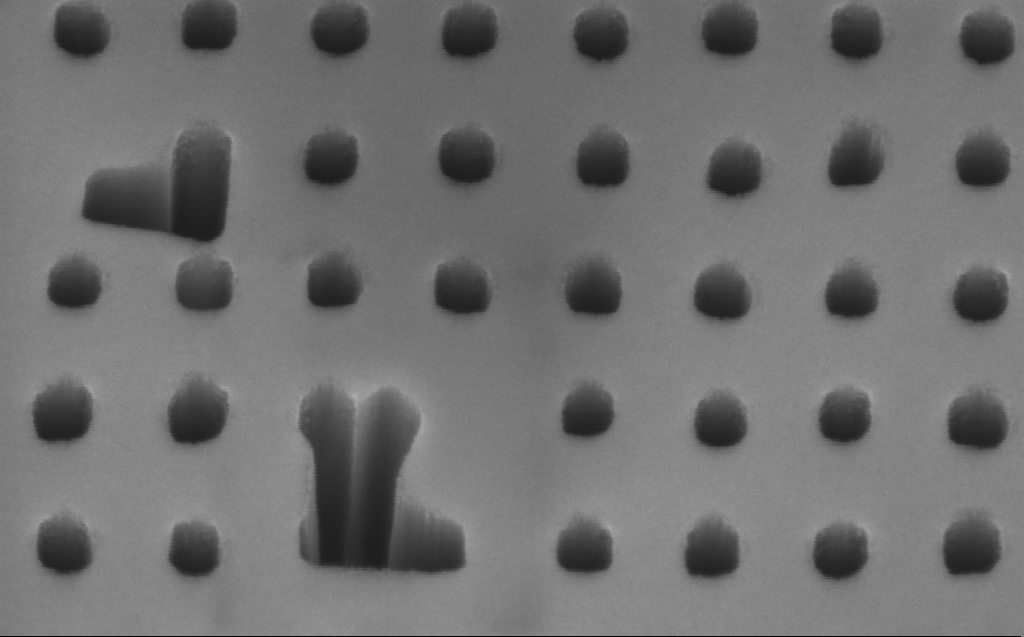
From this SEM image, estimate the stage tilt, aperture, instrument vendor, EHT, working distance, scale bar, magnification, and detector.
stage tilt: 45°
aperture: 30 µm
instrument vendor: Zeiss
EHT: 3 kV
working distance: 6 mm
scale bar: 200 nm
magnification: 96.46 K X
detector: InLens